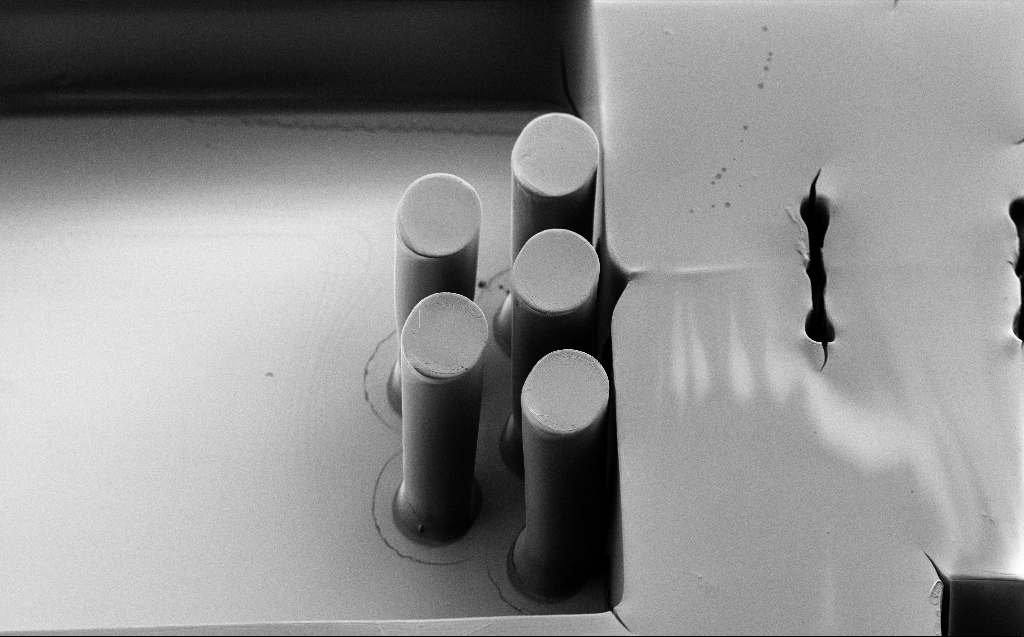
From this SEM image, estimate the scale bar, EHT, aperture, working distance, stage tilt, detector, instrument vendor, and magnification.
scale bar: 20000 nm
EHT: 1.7 kV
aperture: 30 µm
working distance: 8 mm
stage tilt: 45°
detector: SE2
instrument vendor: Zeiss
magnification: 0.748 K X